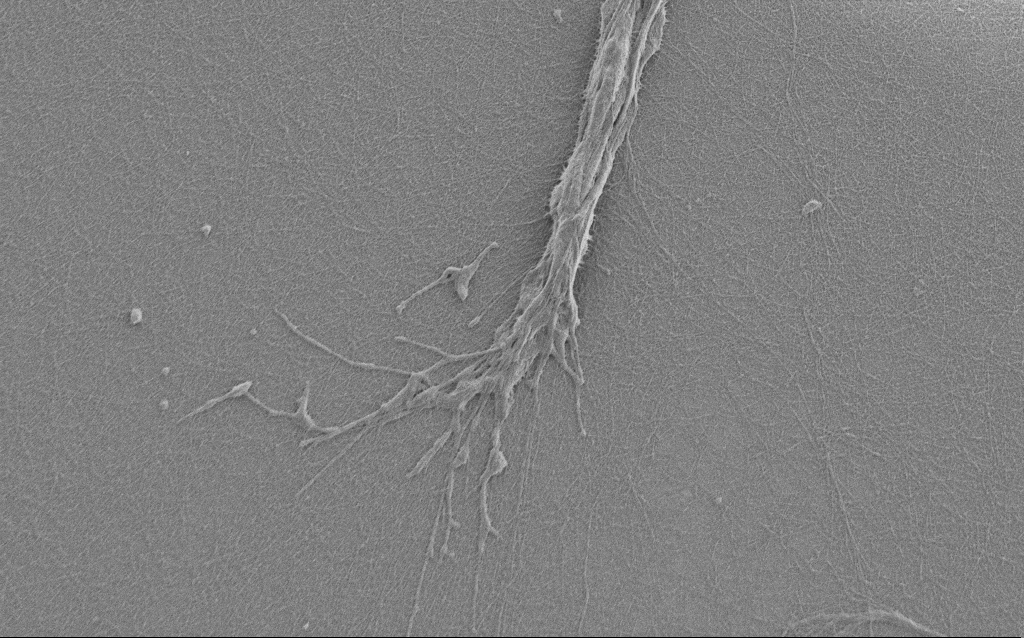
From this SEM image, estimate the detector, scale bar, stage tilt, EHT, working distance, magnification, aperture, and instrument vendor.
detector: SE2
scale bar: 2000 nm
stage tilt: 0°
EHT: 1 kV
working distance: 6 mm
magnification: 7.5 K X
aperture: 30 µm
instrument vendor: Zeiss